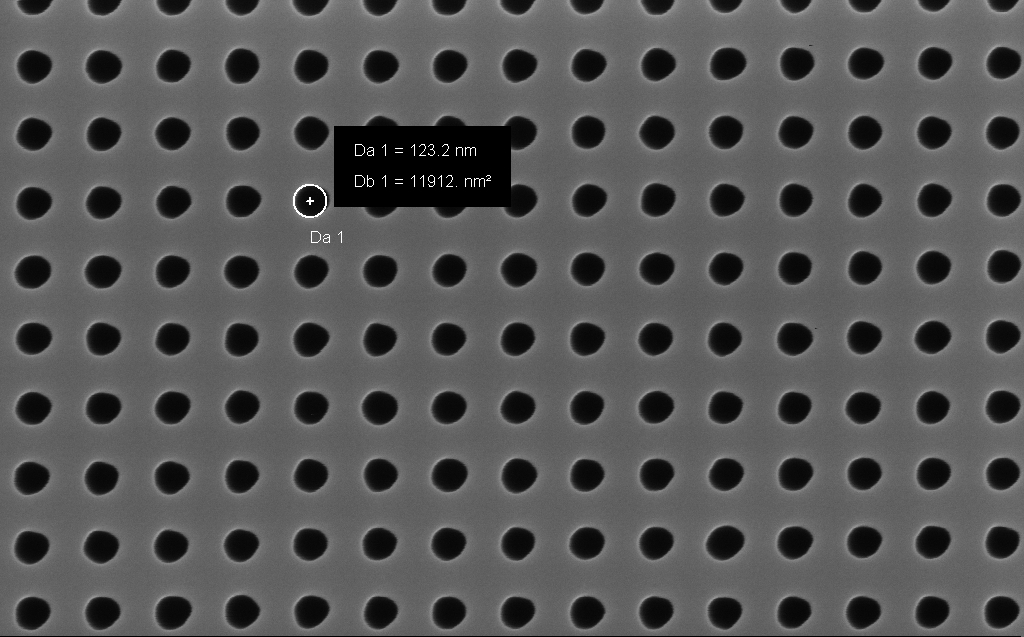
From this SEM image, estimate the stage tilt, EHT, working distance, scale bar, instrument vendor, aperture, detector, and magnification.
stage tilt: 0°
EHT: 5 kV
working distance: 7 mm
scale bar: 200 nm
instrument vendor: Zeiss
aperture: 30 µm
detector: InLens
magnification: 101.37 K X